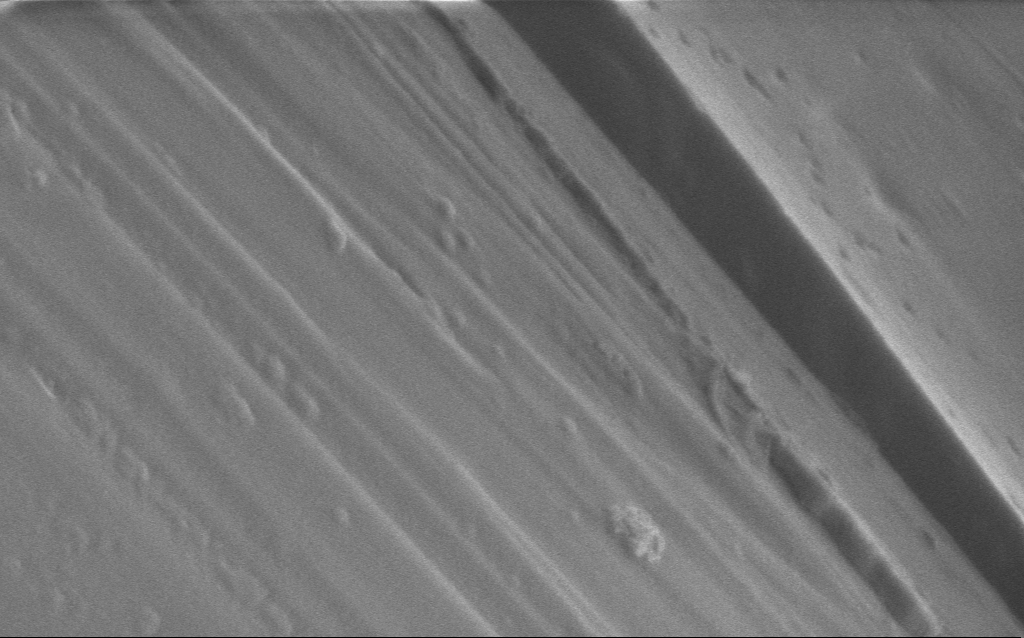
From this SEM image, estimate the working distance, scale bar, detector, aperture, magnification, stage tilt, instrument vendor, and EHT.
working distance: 4 mm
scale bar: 1000 nm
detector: InLens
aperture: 30 µm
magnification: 39.36 K X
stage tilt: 0°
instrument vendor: Zeiss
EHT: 1 kV